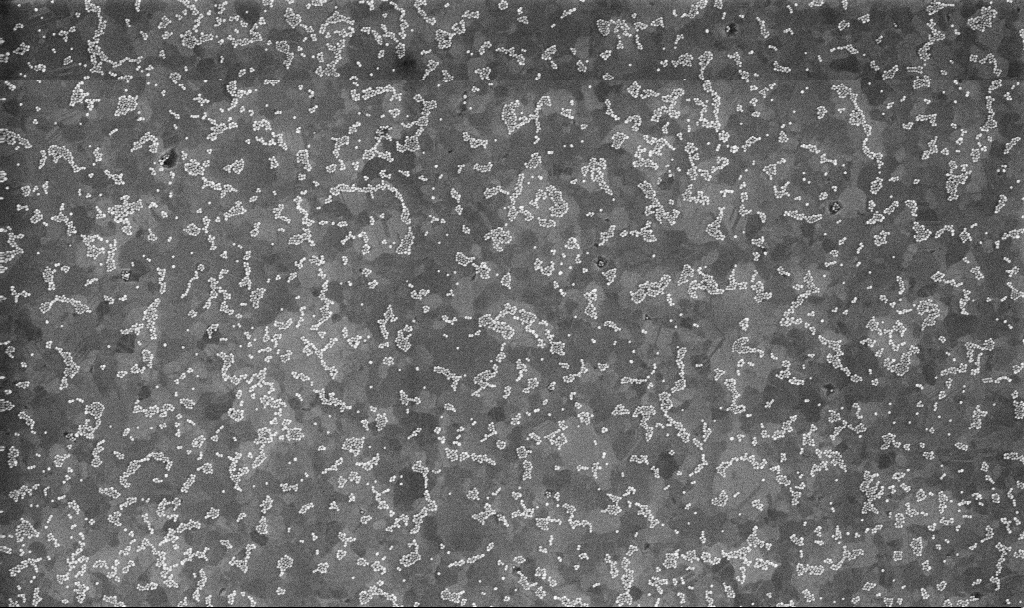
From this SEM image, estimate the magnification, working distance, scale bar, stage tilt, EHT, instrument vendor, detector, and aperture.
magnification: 50 K X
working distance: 3.3 mm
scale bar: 1000 nm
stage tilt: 0°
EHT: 10 kV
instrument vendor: Zeiss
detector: InLens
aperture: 30 µm